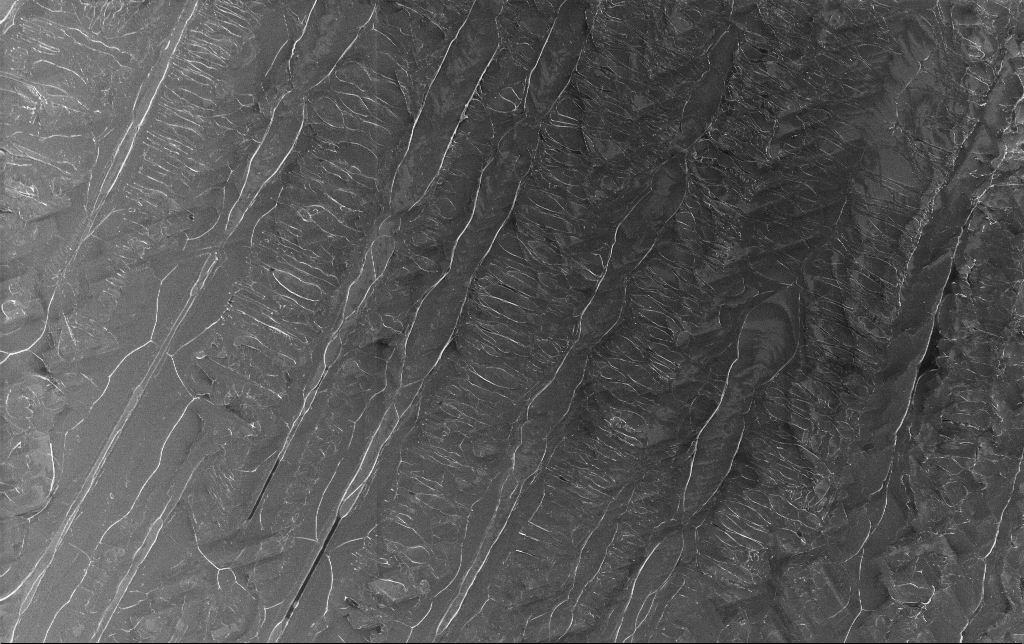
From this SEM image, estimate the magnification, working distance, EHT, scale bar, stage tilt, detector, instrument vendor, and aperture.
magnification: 0.697 K X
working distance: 3.1 mm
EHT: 5 kV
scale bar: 100000 nm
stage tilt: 0°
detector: InLens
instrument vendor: Zeiss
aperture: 30 µm